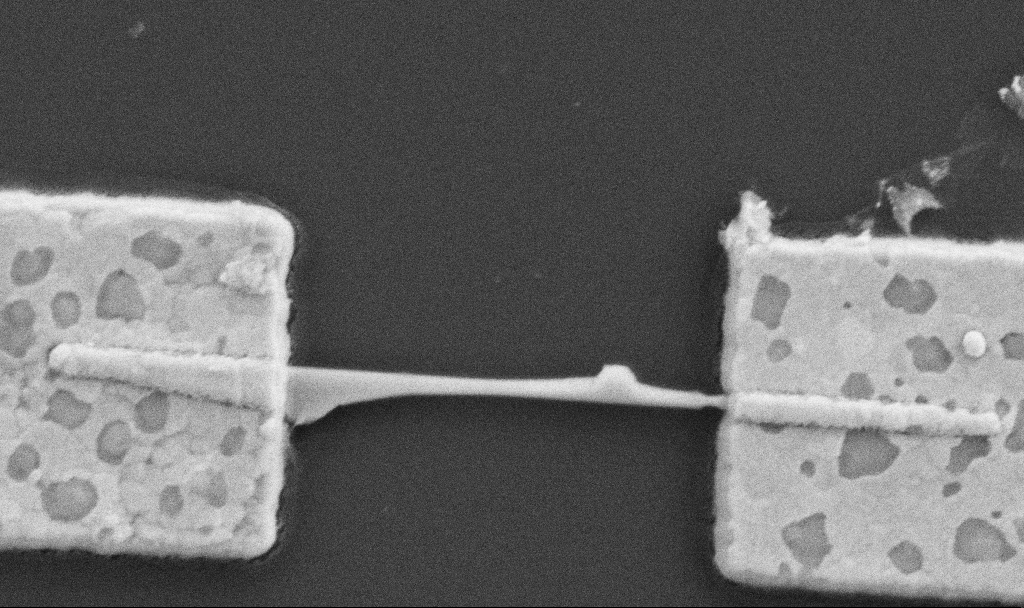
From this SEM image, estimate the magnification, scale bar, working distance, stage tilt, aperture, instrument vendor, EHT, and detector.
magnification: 60 K X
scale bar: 1000 nm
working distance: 10.7 mm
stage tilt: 0°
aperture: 30 µm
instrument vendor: Zeiss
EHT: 5 kV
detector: SE2